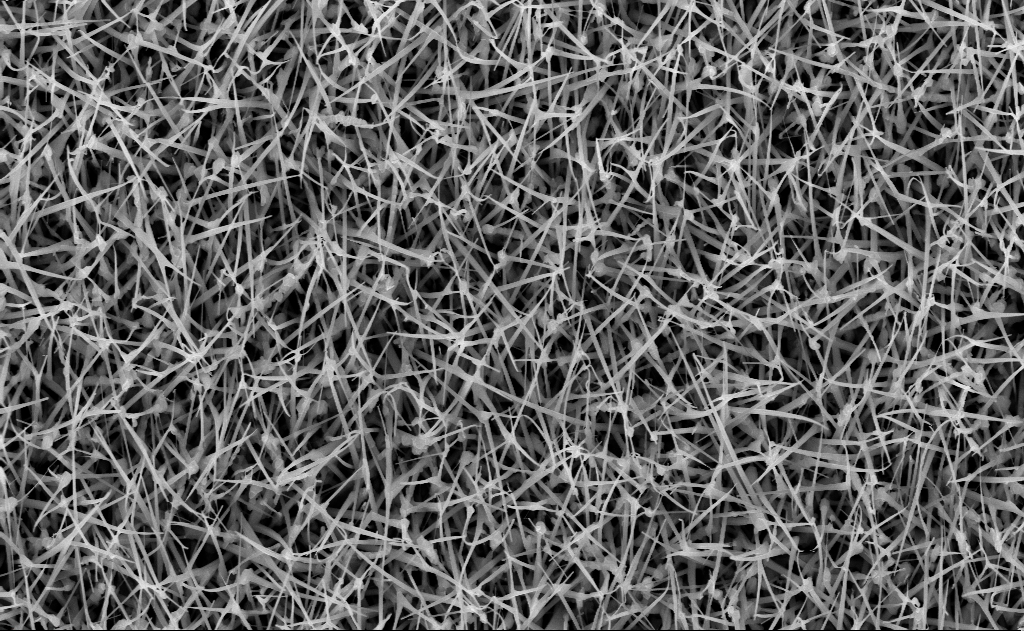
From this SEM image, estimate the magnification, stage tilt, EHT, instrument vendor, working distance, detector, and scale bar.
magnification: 20 K X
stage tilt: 0°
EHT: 10 kV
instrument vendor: Zeiss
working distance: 10 mm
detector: InLens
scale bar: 2000 nm